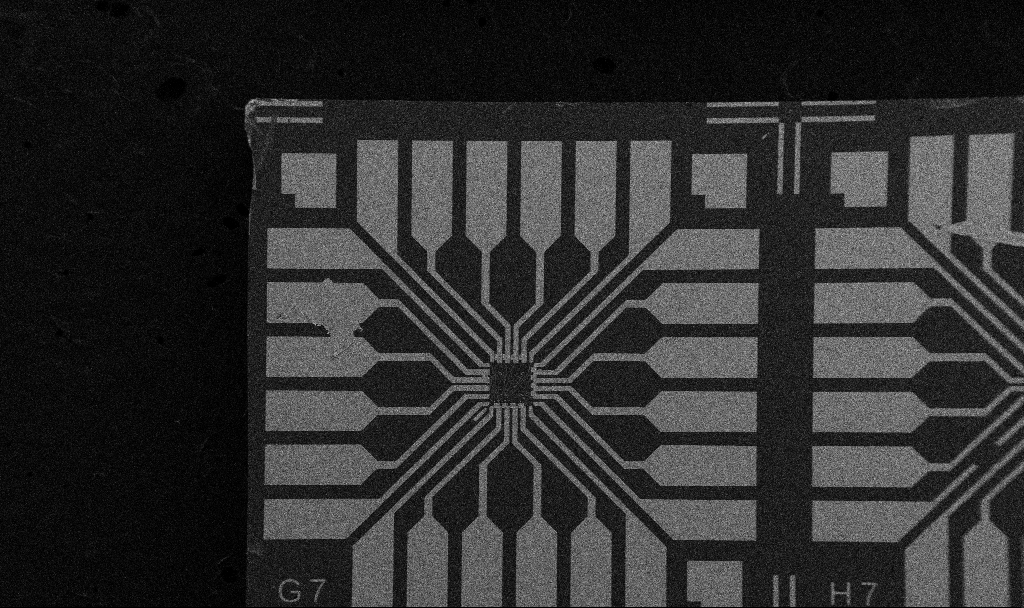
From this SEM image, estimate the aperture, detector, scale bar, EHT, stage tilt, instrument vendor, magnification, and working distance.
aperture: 30 µm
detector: SE2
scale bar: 200000 nm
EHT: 5 kV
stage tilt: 0°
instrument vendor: Zeiss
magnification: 0.1 K X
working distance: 10.7 mm